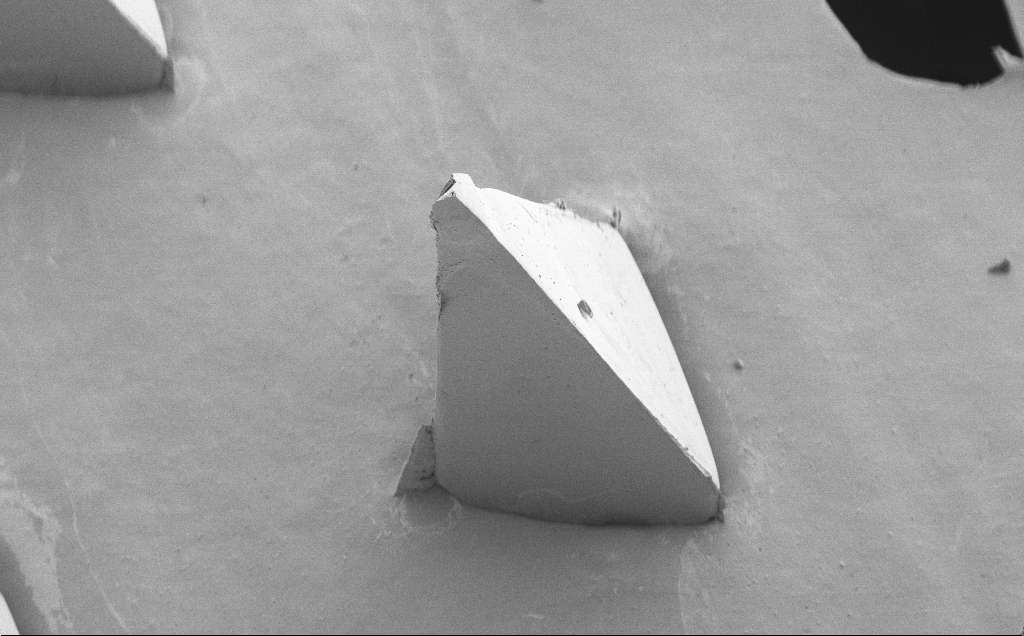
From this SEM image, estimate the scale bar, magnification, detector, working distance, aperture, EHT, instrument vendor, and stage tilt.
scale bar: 200000 nm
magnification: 0.172 K X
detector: SE2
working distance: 8 mm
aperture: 30 µm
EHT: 5 kV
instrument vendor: Zeiss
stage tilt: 40°